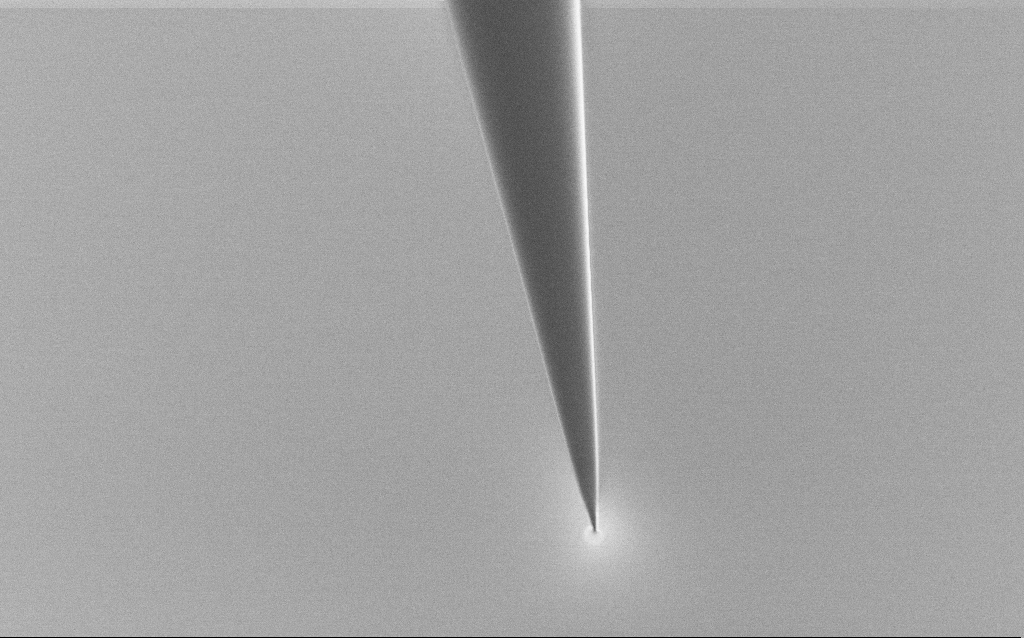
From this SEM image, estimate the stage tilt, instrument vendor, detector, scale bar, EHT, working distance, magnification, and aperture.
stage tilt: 45°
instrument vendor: Zeiss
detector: SE2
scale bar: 10000 nm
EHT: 1 kV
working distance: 6 mm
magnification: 5 K X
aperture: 30 µm